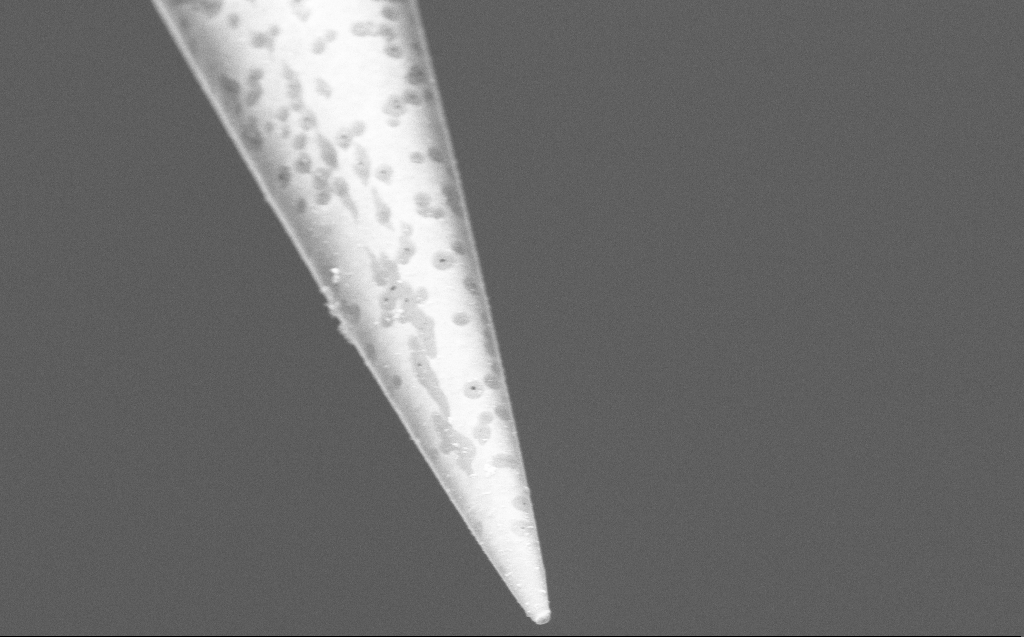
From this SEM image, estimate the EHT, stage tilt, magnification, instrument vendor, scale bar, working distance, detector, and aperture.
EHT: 5 kV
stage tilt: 45°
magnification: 50 K X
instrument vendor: Zeiss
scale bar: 1000 nm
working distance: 6 mm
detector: InLens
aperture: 30 µm